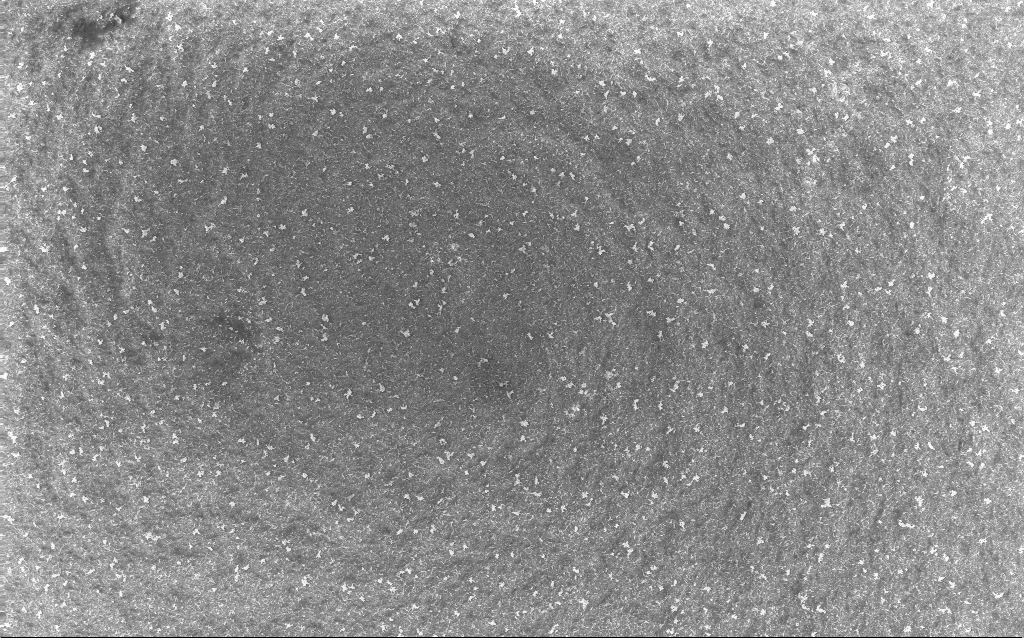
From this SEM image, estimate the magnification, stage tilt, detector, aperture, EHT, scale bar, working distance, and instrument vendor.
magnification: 1 K X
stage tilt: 0°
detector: InLens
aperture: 30 µm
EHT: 10 kV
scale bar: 20000 nm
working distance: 2.5 mm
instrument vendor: Zeiss